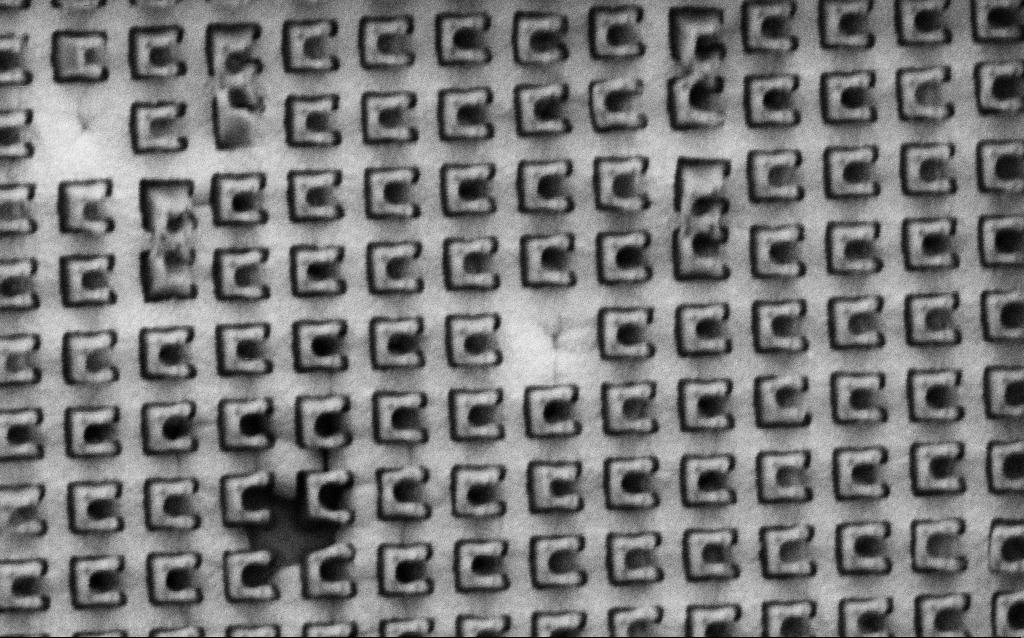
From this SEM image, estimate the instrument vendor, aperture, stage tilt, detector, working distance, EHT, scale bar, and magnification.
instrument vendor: Zeiss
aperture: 30 µm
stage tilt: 0°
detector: SE2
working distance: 8 mm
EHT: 1.5 kV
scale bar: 1000 nm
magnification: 60.3 K X